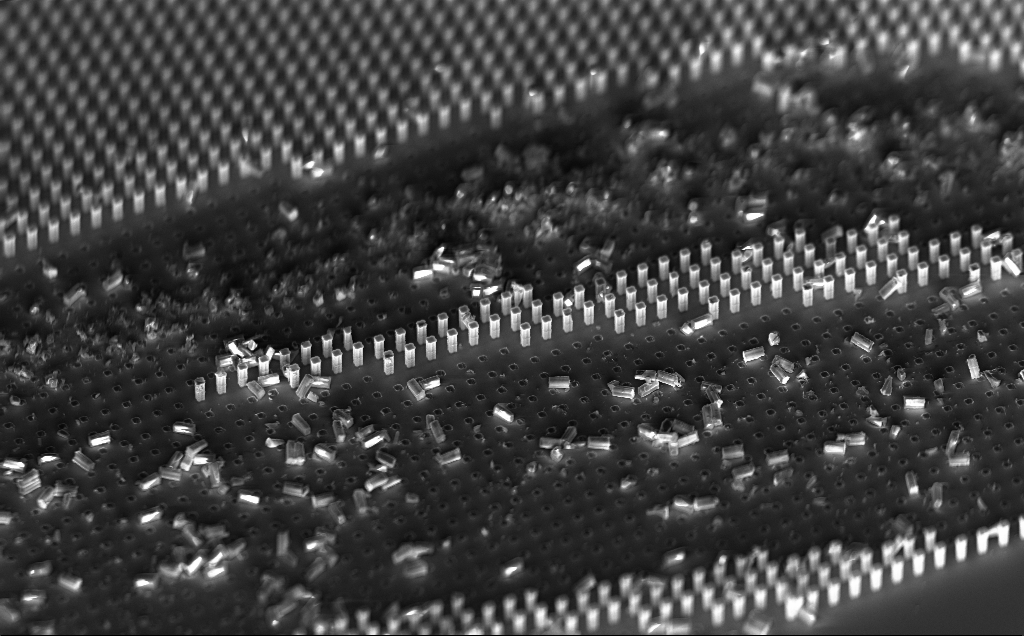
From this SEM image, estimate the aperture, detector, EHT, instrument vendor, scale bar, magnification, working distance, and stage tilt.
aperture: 120 µm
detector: InLens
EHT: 10 kV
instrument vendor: Zeiss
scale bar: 20000 nm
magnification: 0.853 K X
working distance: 10 mm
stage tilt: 48.7°